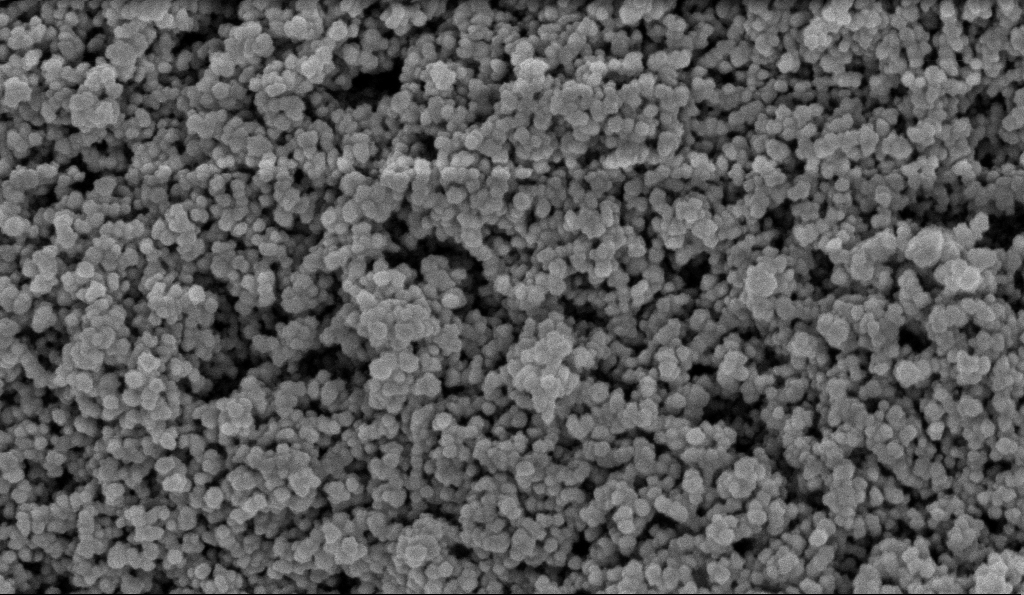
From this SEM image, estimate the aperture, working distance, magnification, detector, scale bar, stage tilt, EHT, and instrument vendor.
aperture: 30 µm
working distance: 5.9 mm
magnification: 135 K X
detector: InLens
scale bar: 200 nm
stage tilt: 0°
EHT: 5 kV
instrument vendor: Zeiss